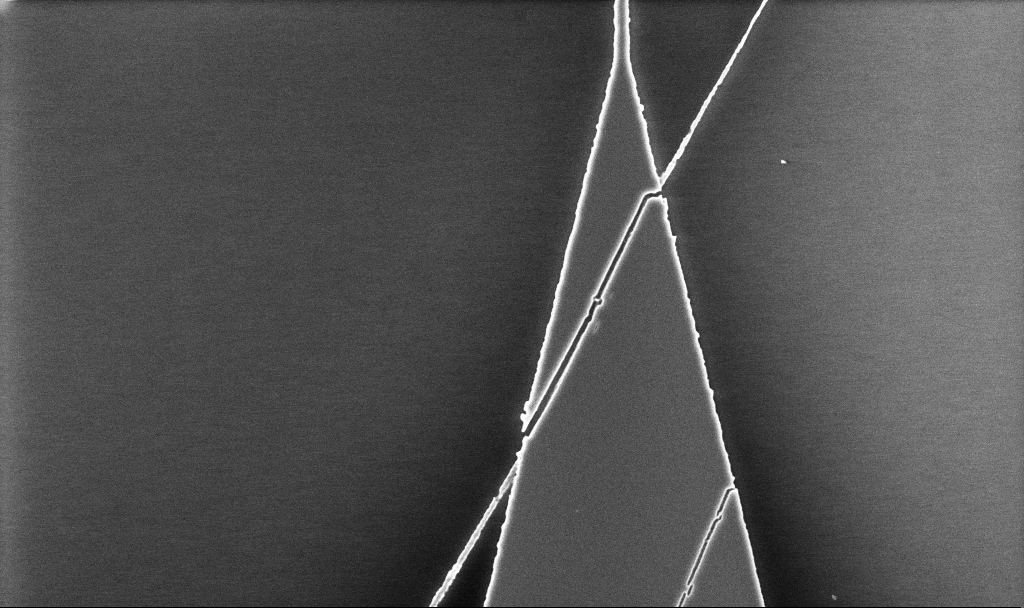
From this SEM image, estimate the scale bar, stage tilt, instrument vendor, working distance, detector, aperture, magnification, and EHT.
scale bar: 2000 nm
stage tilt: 0°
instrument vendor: Zeiss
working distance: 5.2 mm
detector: InLens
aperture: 30 µm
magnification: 9.91 K X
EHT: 5 kV